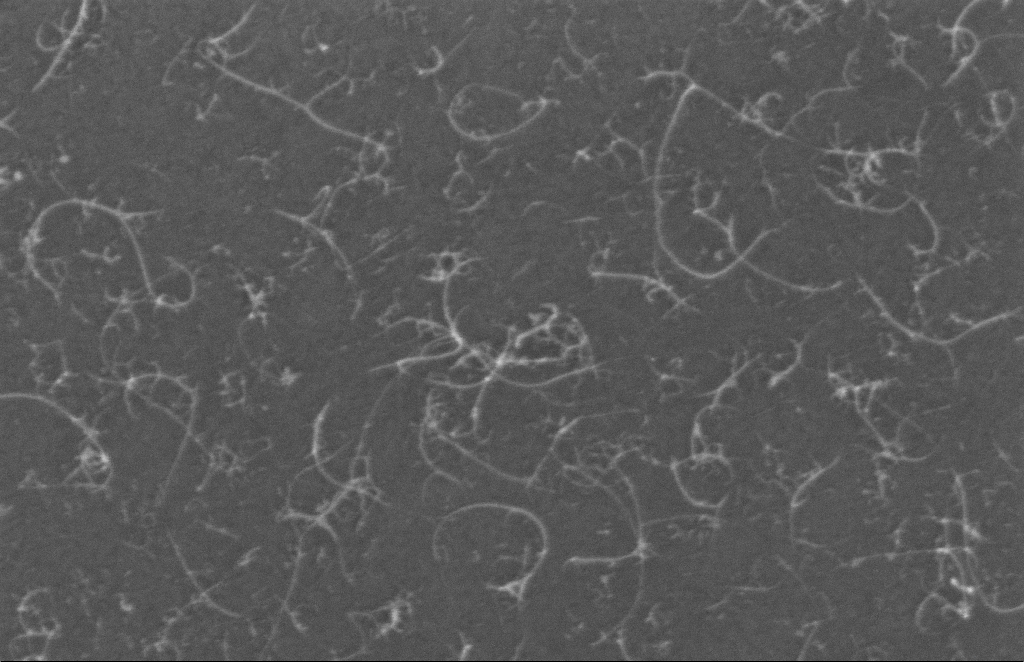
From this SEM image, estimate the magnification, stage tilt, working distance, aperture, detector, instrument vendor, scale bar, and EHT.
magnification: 242.02 K X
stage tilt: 0°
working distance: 8 mm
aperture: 20 µm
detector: InLens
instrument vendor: Zeiss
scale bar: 100 nm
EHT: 5 kV